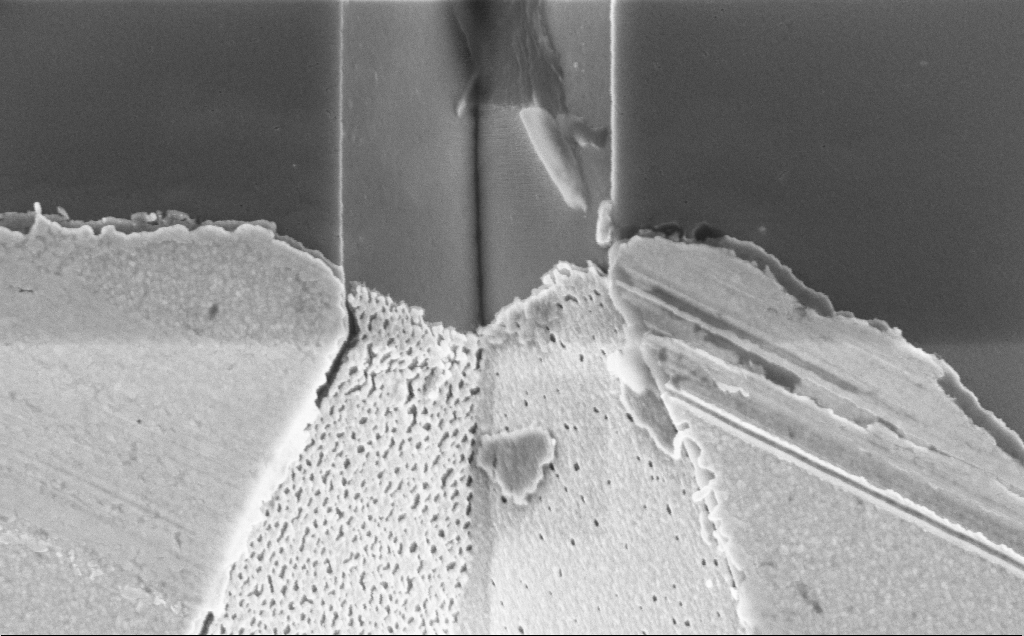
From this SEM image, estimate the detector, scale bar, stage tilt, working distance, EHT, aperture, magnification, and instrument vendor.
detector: InLens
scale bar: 1000 nm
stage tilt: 0.2°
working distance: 15 mm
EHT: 5 kV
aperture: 30 µm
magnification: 47.56 K X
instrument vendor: Zeiss